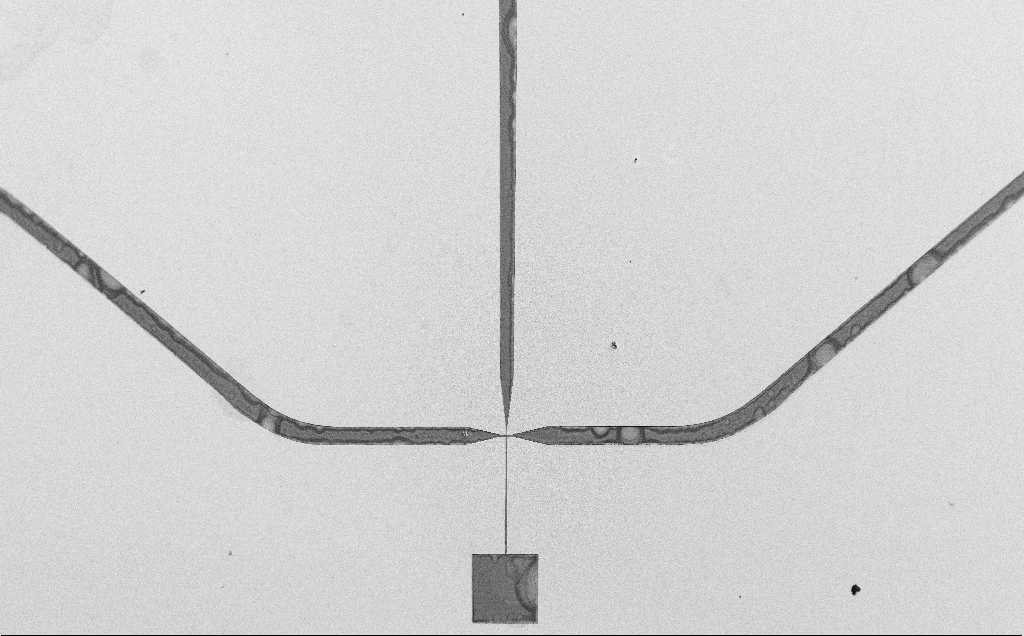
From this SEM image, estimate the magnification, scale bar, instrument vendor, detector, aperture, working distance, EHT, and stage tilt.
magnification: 0.061 K X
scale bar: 1e+06 nm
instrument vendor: Zeiss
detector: SE2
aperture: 30 µm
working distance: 14 mm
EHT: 10 kV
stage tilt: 0°